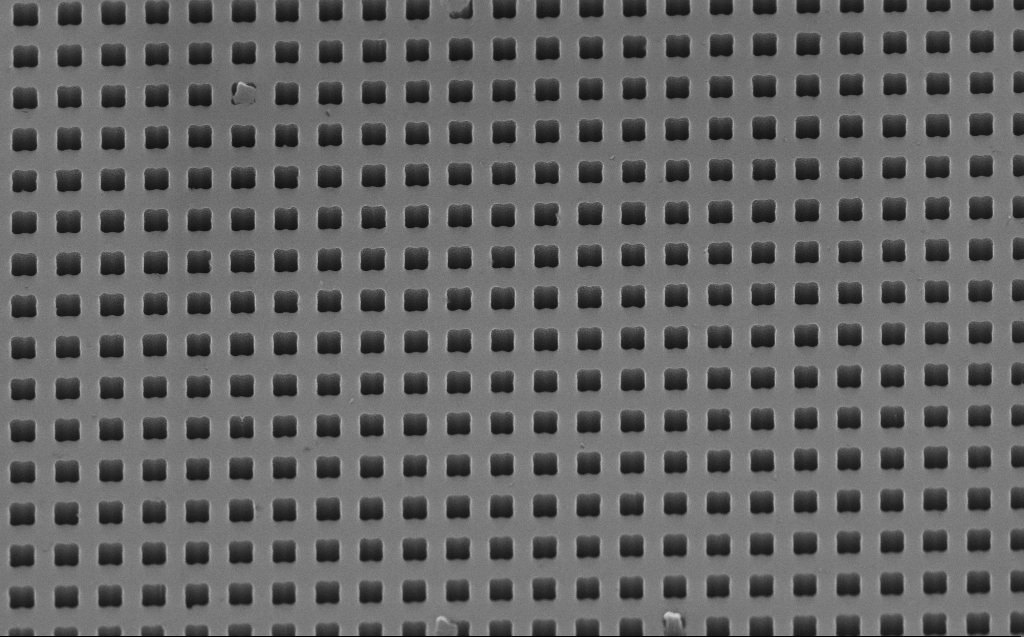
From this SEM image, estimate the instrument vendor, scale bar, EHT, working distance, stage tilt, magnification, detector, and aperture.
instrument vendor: Zeiss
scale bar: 1000 nm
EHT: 10 kV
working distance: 7 mm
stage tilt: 45°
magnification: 32.22 K X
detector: InLens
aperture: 30 µm